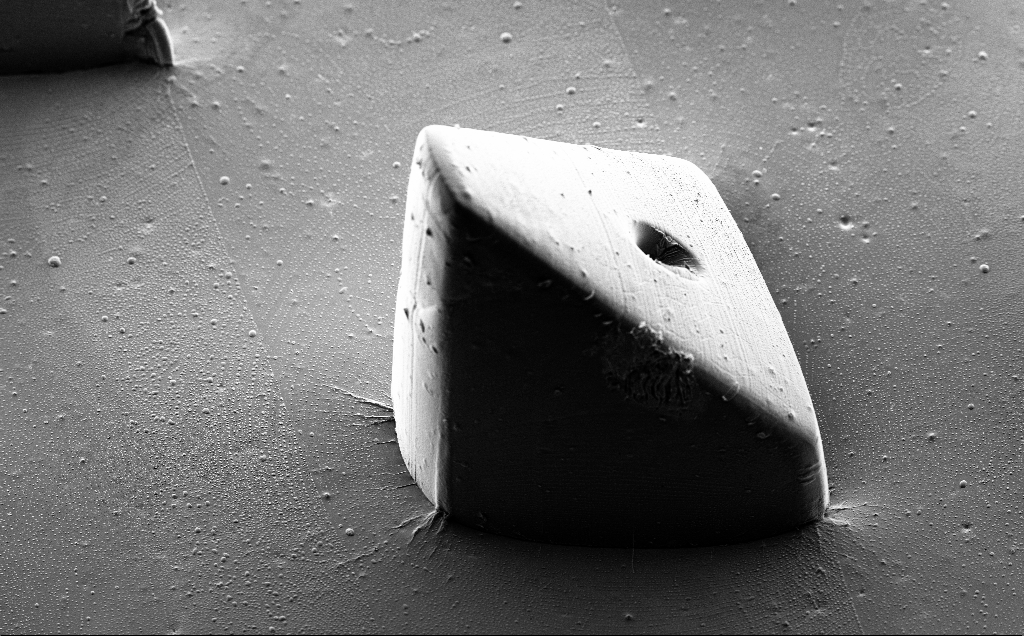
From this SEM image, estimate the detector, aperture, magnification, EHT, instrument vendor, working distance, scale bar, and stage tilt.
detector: SE2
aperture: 30 µm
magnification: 0.366 K X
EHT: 5 kV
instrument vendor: Zeiss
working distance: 9 mm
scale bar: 100000 nm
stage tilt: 35°